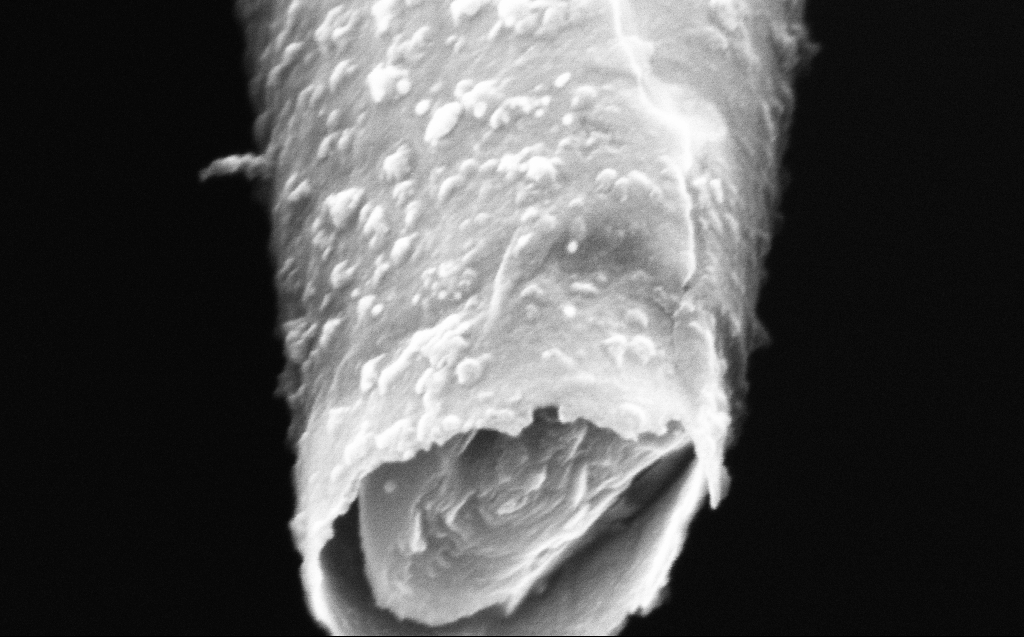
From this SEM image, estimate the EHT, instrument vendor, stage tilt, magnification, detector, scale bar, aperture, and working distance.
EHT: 4 kV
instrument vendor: Zeiss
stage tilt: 45°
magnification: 250 K X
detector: InLens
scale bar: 200 nm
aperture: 30 µm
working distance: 4 mm